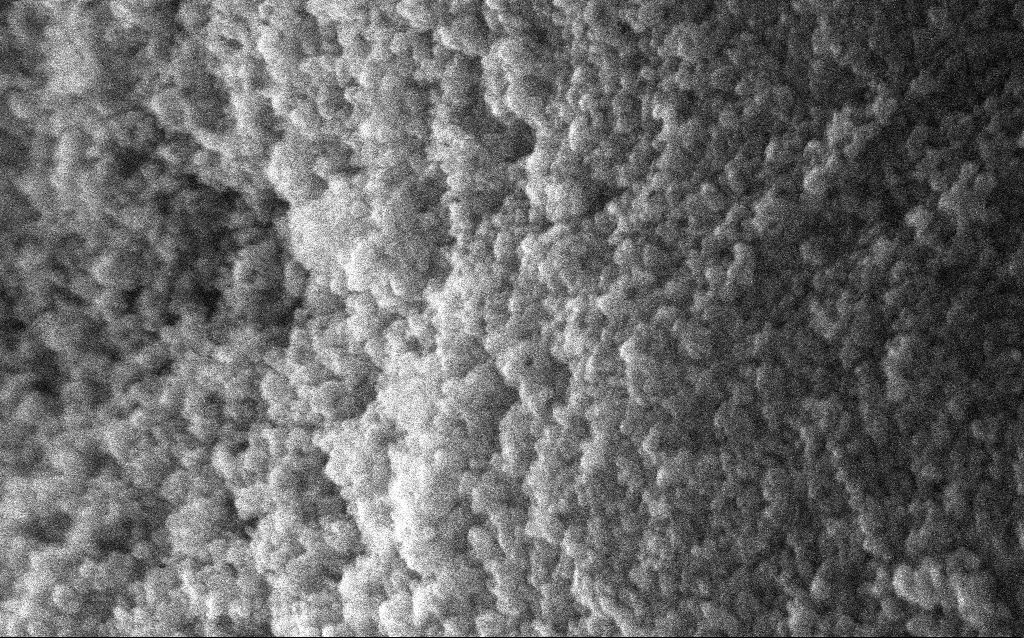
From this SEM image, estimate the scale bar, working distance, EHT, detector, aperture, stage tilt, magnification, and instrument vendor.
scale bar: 100 nm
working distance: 2.7 mm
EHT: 10 kV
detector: InLens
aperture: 30 µm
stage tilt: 0°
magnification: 204.13 K X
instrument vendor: Zeiss